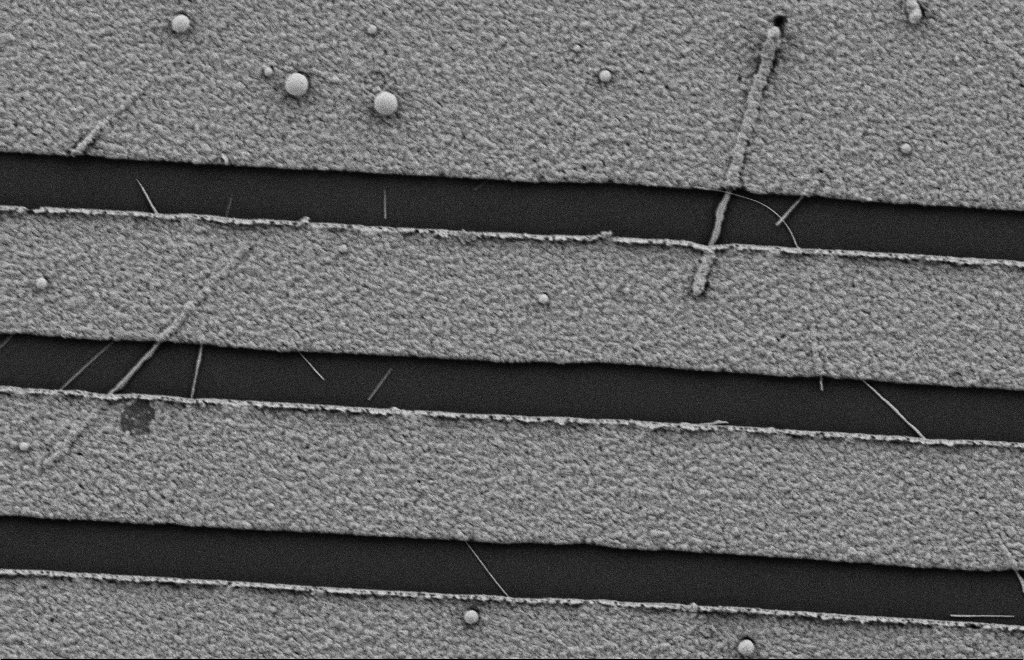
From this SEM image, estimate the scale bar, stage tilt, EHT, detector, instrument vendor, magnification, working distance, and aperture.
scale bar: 2000 nm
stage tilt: -0.3°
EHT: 2 kV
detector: SE2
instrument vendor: Zeiss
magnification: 16.65 K X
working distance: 10 mm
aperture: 20 µm